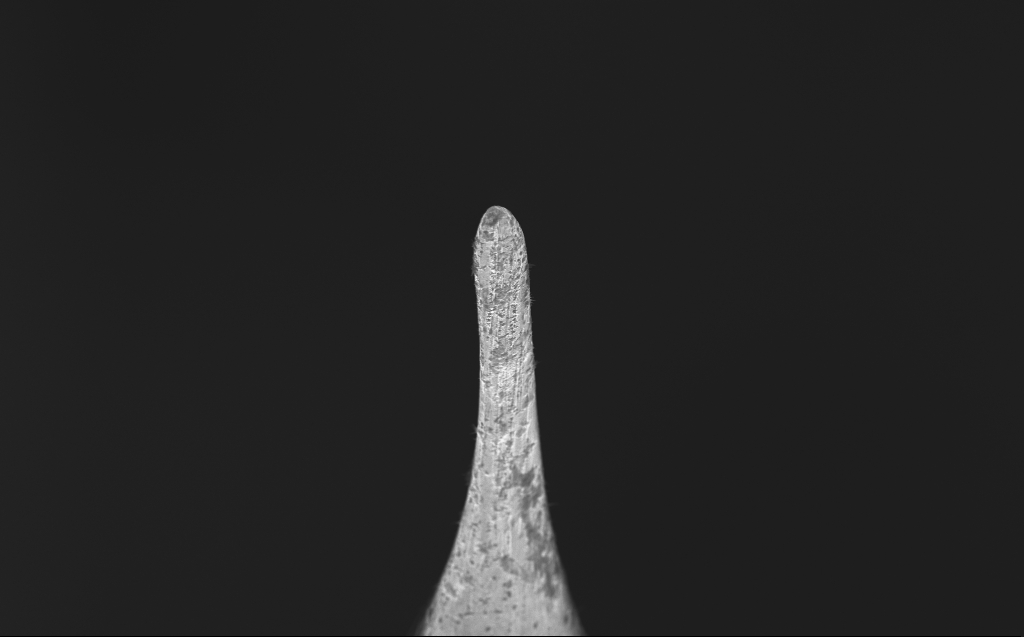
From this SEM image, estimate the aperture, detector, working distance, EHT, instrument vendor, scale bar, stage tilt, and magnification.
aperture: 30 µm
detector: InLens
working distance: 4 mm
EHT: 10 kV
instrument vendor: Zeiss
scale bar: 20000 nm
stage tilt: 40°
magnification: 2.61 K X